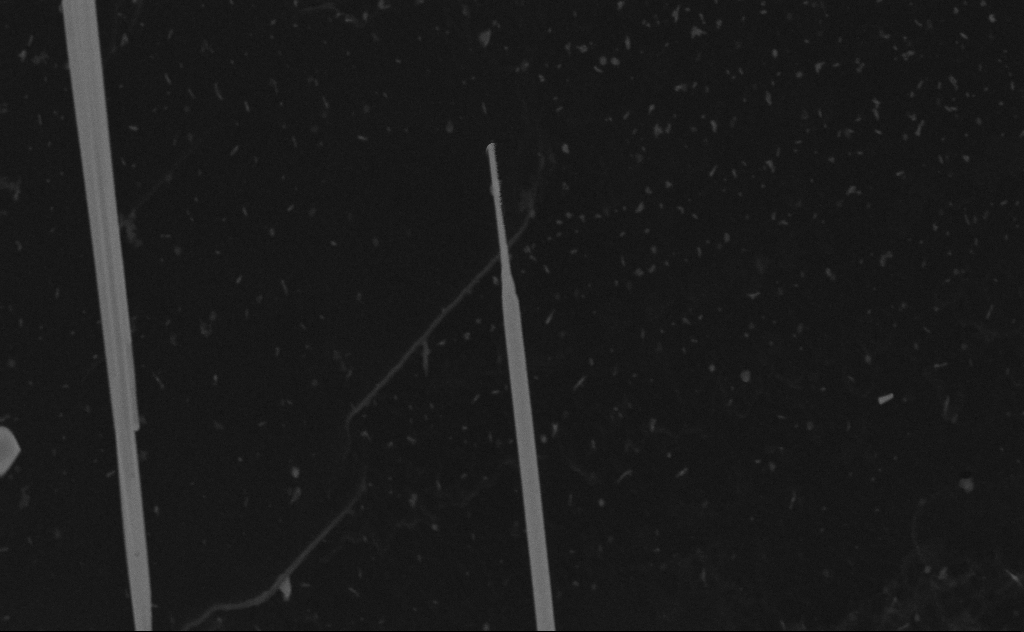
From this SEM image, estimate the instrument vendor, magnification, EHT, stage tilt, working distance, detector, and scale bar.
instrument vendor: Zeiss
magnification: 103.14 K X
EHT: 20 kV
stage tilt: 0°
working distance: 8 mm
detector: SE2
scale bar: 200 nm